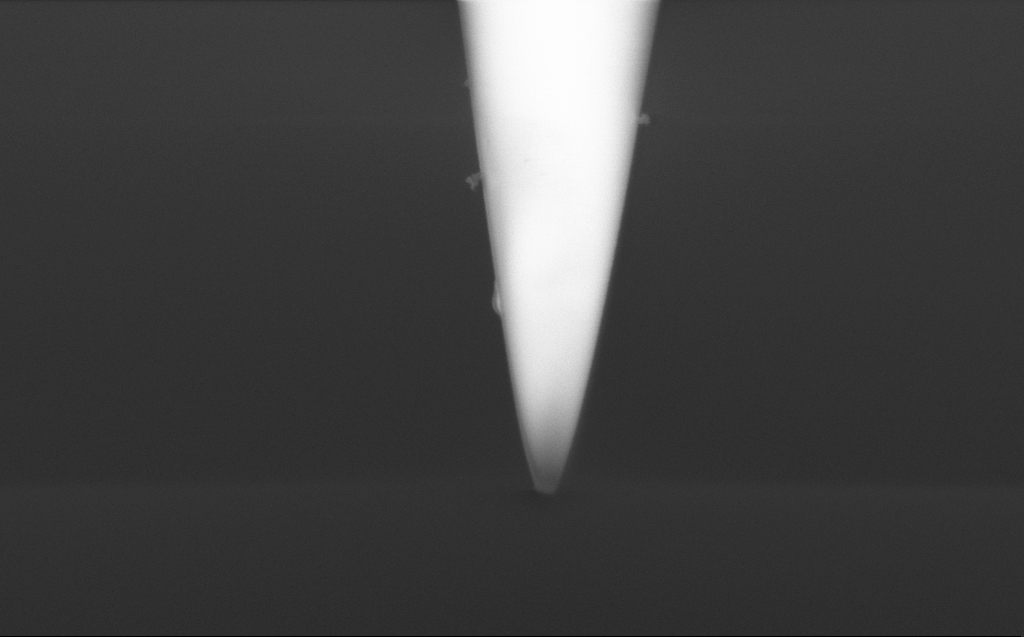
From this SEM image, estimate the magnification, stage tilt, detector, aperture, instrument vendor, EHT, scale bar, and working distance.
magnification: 90.26 K X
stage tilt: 45.1°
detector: InLens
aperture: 30 µm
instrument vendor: Zeiss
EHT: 2 kV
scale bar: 200 nm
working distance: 3 mm